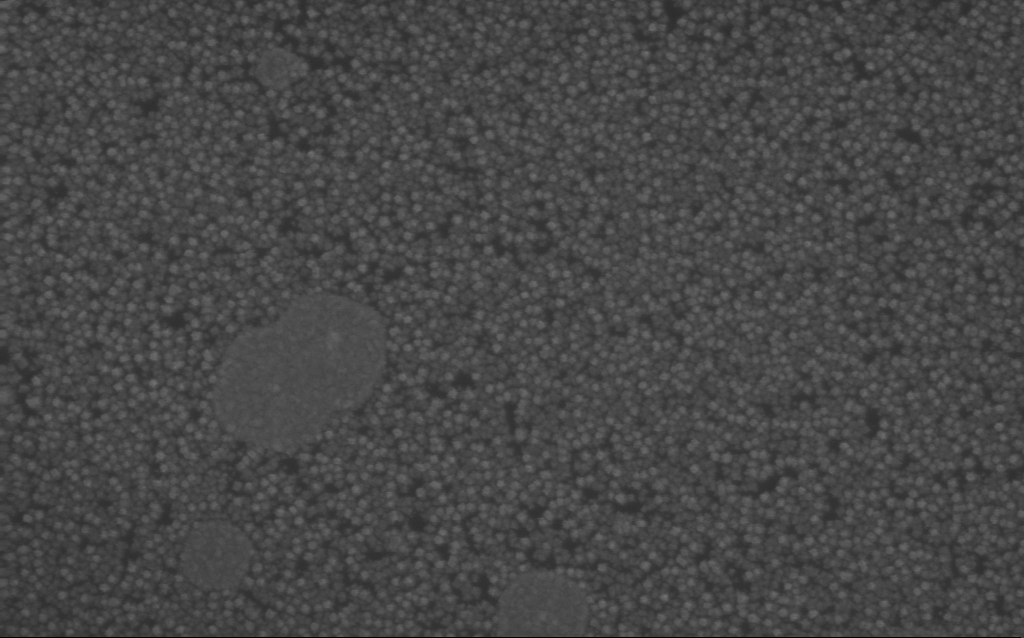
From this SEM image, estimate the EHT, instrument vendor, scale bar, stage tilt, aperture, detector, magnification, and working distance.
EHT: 7 kV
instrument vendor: Zeiss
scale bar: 200 nm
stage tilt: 0°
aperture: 30 µm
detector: InLens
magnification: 220.66 K X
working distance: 3 mm